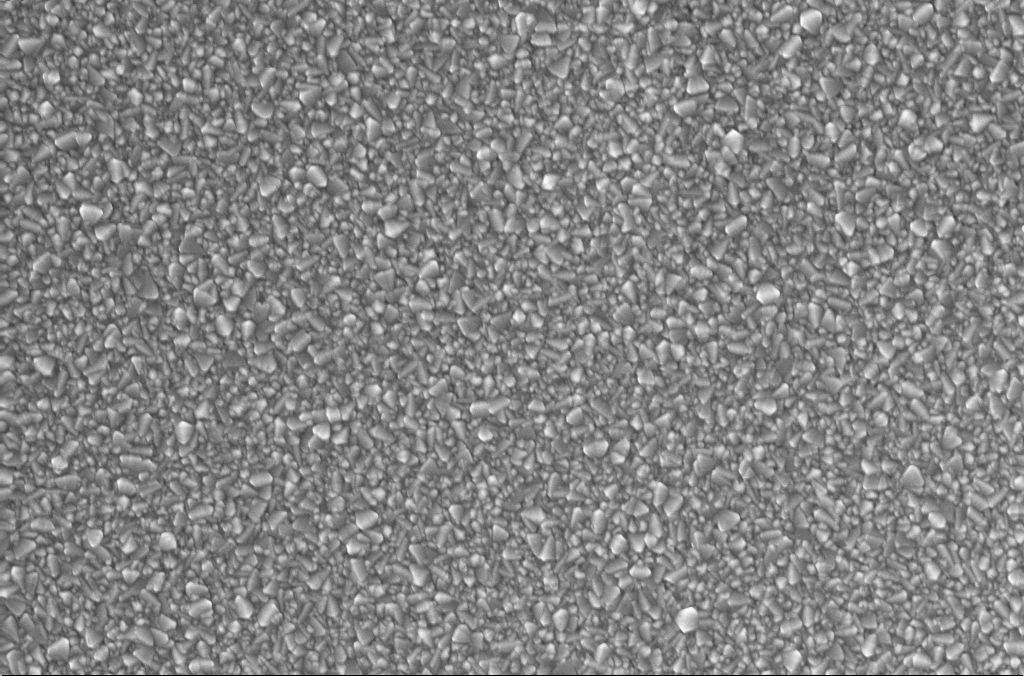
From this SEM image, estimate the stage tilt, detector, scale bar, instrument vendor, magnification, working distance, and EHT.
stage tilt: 0°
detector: InLens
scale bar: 2000 nm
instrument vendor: Zeiss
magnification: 20 K X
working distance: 1.9 mm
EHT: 10 kV